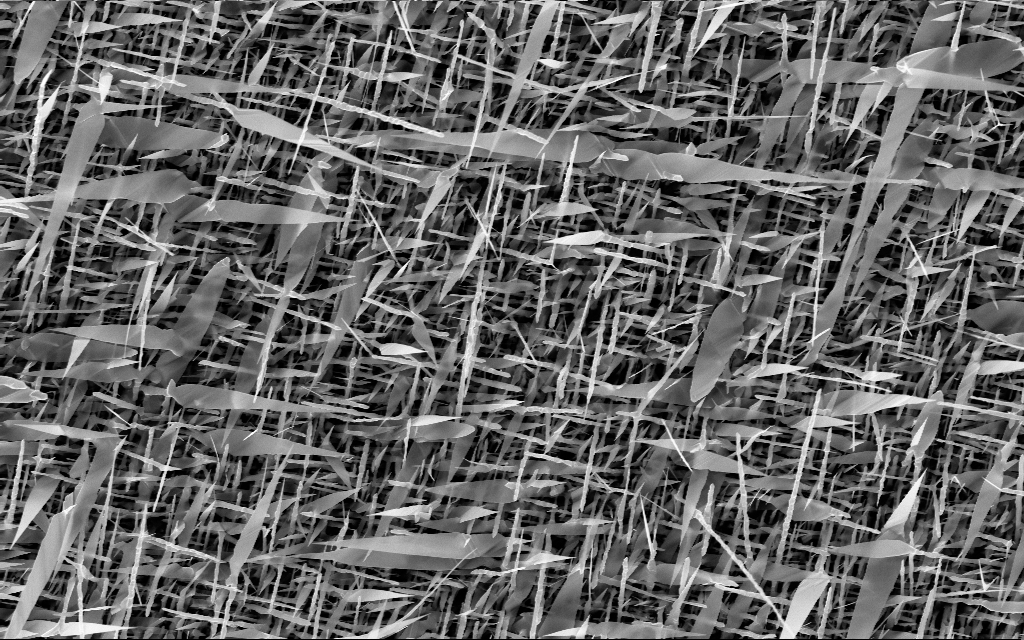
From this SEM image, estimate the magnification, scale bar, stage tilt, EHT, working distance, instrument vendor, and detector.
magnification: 10 K X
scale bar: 2000 nm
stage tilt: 0°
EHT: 10 kV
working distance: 7 mm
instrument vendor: Zeiss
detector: InLens